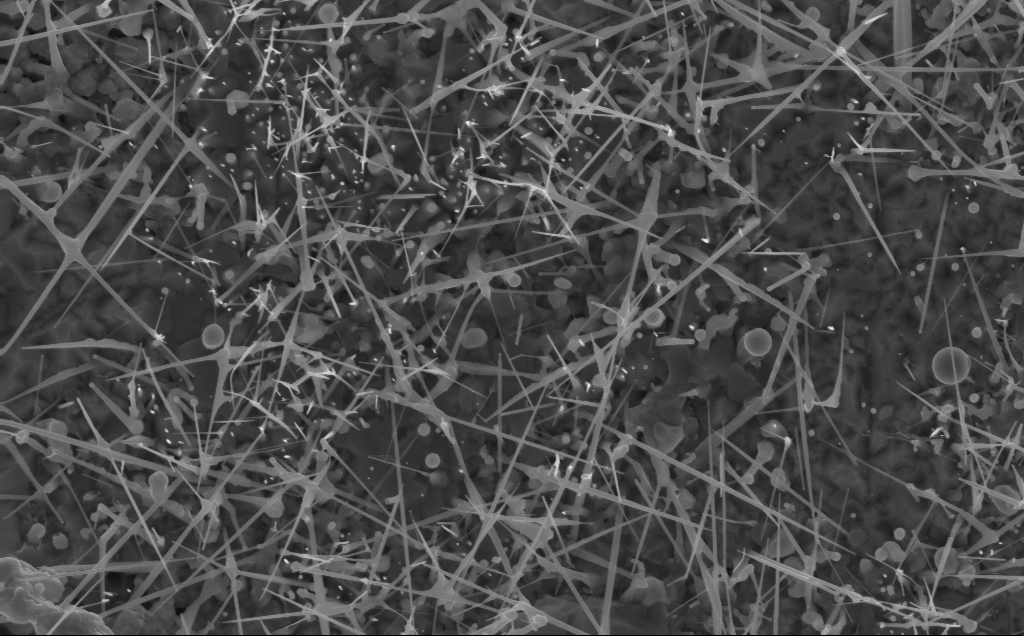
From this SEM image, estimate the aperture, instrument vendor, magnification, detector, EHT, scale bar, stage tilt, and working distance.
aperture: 30 µm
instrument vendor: Zeiss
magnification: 20 K X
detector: InLens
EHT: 10 kV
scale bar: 2000 nm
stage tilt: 0°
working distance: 3 mm